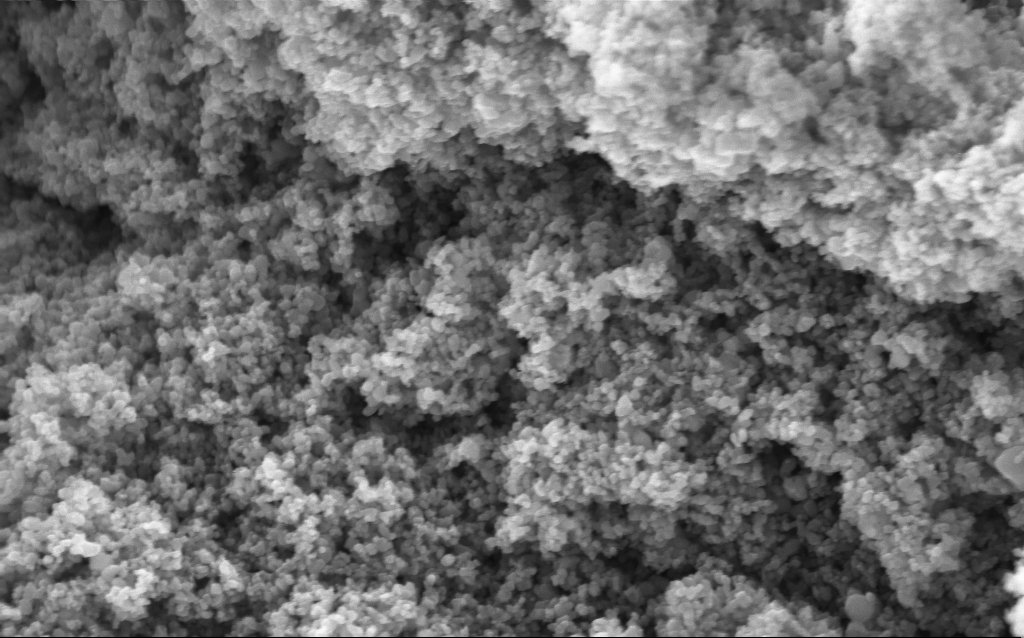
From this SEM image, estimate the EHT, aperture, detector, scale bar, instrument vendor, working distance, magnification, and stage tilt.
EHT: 5 kV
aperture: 30 µm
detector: InLens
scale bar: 200 nm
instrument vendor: Zeiss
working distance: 4.4 mm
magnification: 114.73 K X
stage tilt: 0°